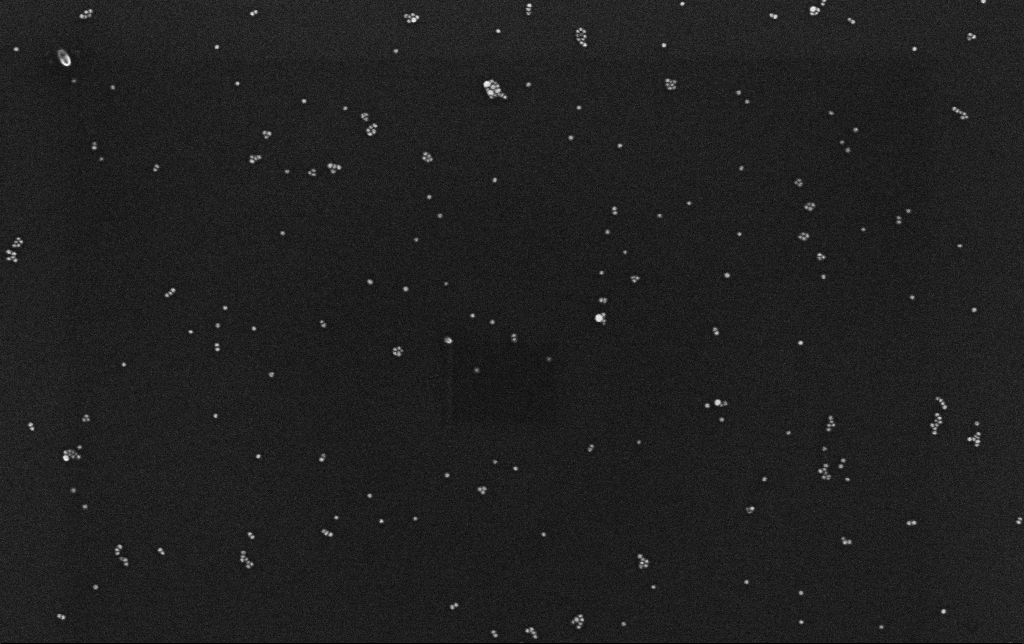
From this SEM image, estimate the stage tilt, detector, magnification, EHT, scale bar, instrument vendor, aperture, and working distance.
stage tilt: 0°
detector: InLens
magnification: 100 K X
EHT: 10 kV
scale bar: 200 nm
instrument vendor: Zeiss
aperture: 30 µm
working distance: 3.1 mm